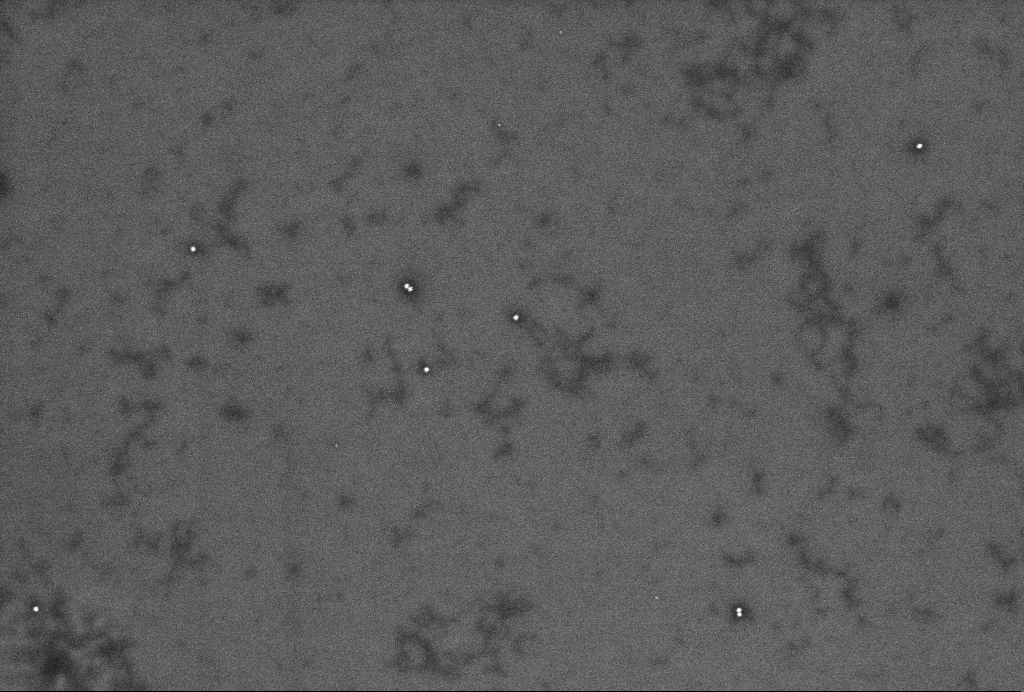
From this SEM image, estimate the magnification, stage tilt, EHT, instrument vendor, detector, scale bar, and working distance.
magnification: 62.39 K X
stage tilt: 0°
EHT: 2 kV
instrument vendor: Zeiss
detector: InLens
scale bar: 200 nm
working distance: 3.3 mm